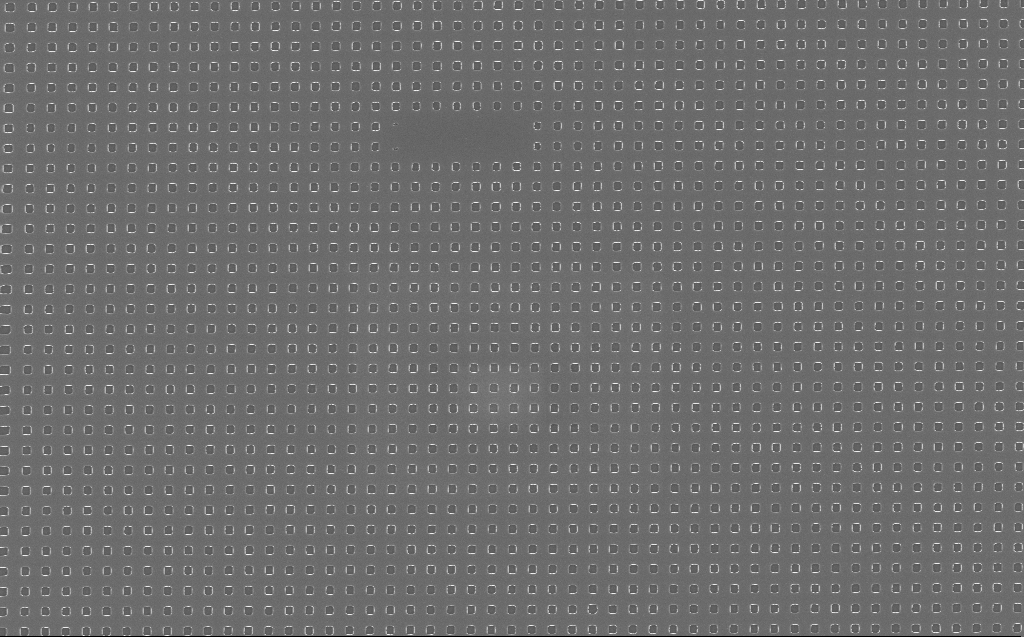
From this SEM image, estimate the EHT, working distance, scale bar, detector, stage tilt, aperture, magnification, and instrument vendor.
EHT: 10 kV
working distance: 7 mm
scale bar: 1000 nm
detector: InLens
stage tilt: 0°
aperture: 30 µm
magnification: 15 K X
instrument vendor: Zeiss